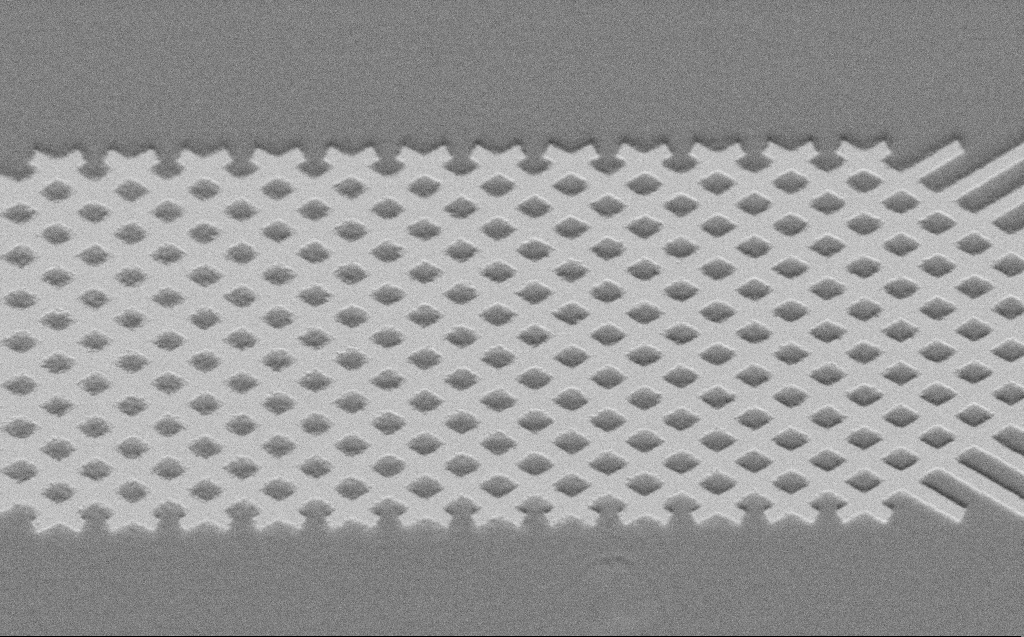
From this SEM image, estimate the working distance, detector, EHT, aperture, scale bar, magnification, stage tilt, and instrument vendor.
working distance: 5 mm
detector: SE2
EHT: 2 kV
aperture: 30 µm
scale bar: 10000 nm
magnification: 5.43 K X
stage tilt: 45°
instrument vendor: Zeiss